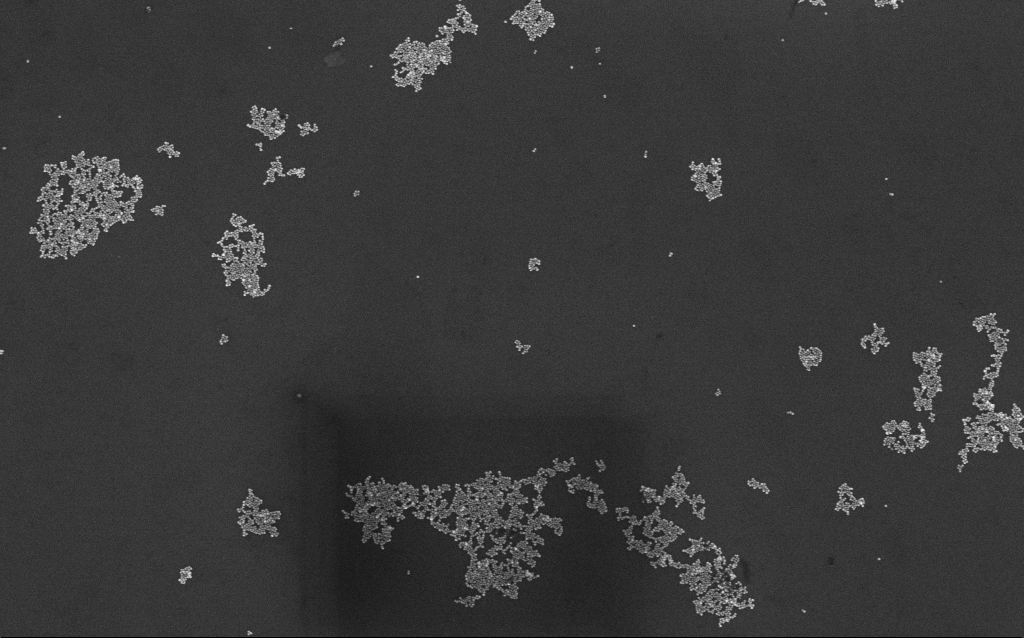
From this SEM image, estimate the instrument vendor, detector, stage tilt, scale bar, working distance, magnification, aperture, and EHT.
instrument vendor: Zeiss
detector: InLens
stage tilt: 0°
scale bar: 1000 nm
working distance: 7 mm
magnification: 50 K X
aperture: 30 µm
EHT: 10 kV